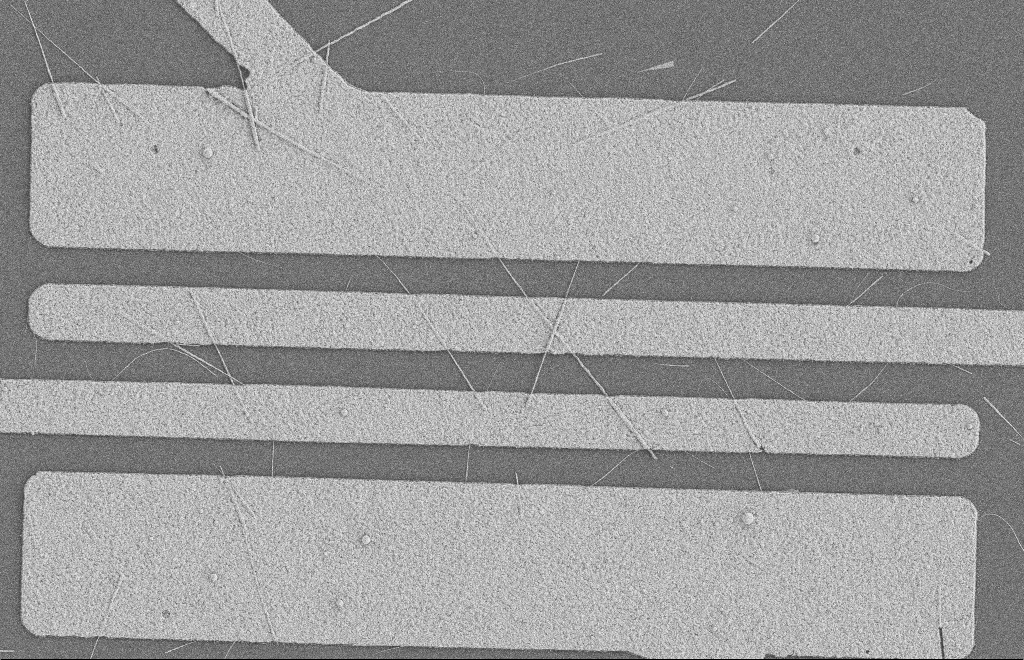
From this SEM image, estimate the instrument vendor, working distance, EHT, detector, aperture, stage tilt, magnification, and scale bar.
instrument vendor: Zeiss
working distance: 8 mm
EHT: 2 kV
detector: SE2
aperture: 20 µm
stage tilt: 0°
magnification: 5.73 K X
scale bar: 2000 nm